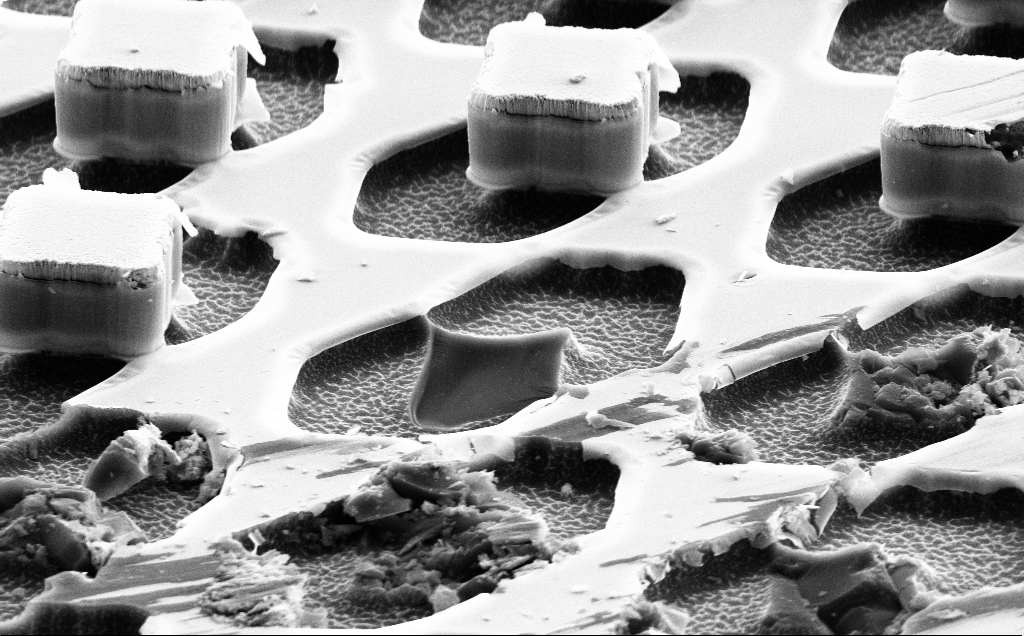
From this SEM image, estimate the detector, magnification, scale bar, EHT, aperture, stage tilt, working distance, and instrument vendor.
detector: SE2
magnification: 7.65 K X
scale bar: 2000 nm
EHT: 10 kV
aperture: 30 µm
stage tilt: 61.7°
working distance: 12 mm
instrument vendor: Zeiss